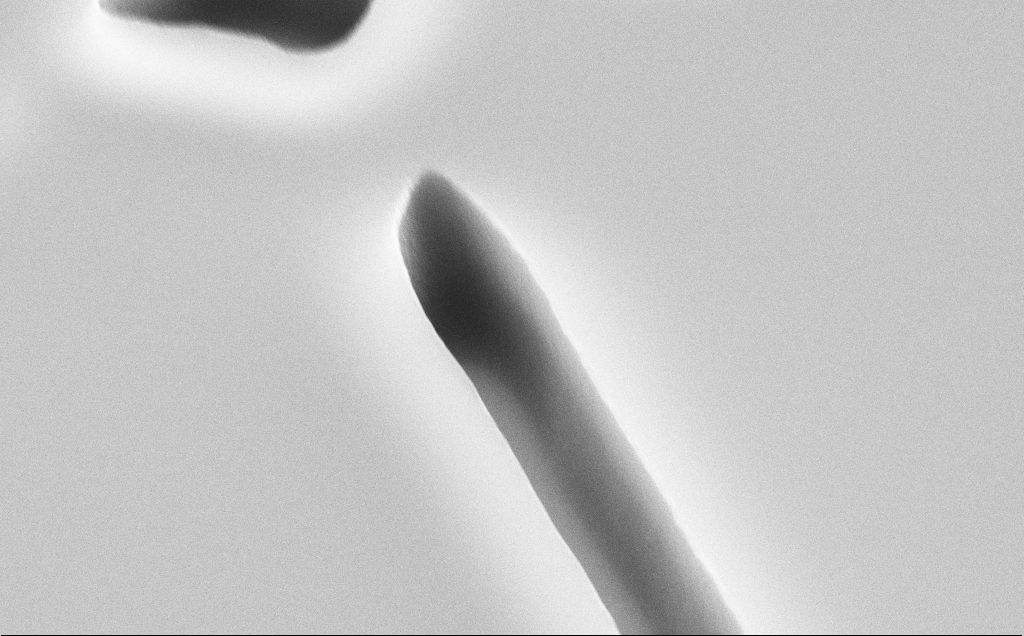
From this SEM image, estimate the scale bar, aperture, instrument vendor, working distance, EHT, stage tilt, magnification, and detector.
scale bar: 2000 nm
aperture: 30 µm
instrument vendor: Zeiss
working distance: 11 mm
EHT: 10 kV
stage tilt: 45°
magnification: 26.98 K X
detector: SE2